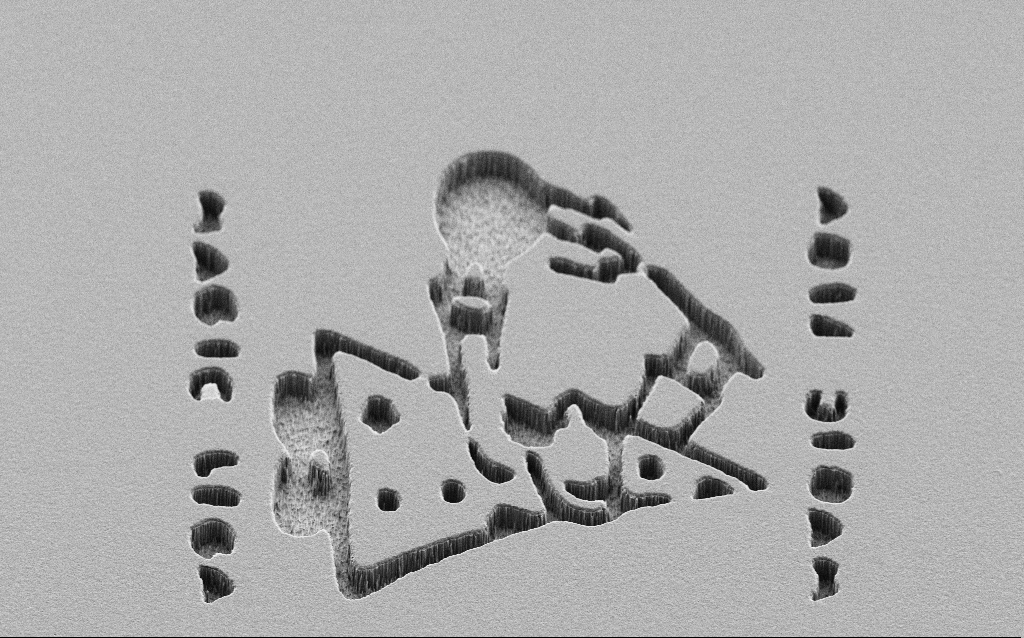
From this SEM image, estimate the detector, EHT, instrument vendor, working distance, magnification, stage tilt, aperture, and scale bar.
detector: SE2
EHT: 3 kV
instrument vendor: Zeiss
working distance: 7 mm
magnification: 1.71 K X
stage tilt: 45°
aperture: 30 µm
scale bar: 20000 nm